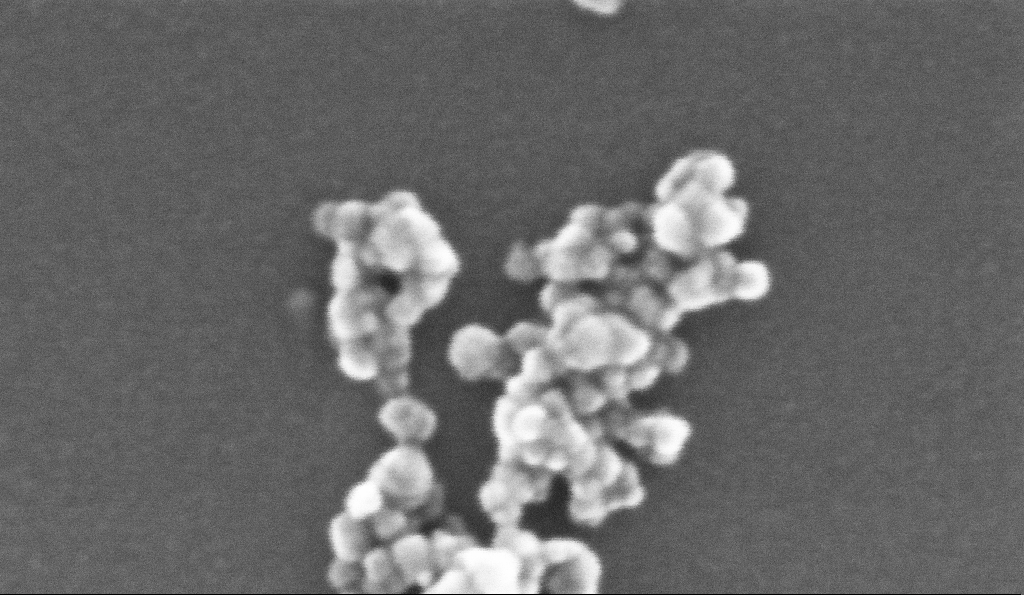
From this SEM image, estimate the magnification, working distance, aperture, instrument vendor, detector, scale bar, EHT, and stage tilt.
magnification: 809.59 K X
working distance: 5.2 mm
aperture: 30 µm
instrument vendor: Zeiss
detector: InLens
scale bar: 20 nm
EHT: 10 kV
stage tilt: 0°